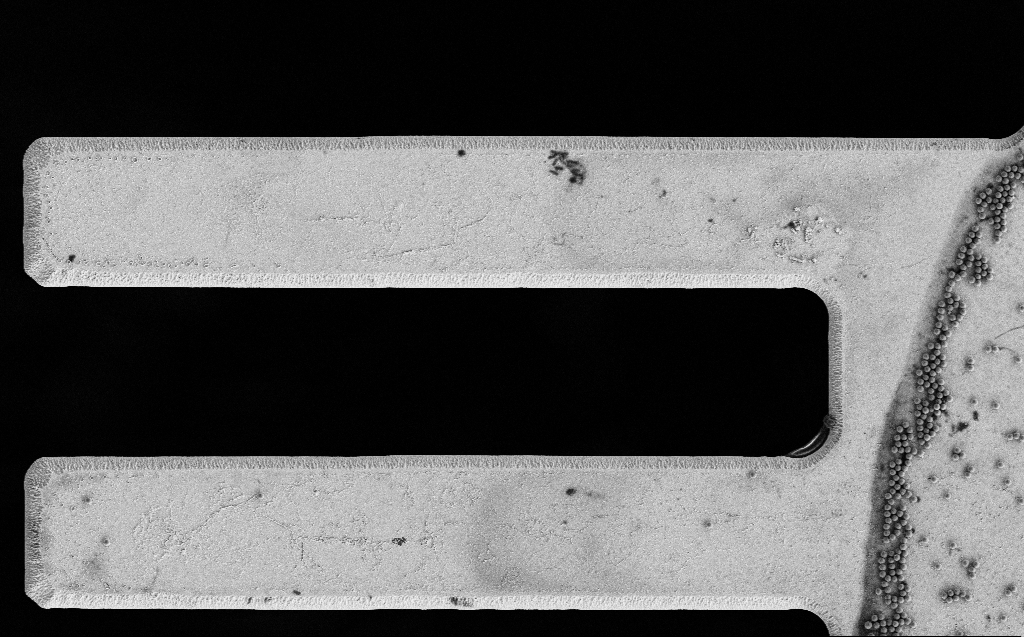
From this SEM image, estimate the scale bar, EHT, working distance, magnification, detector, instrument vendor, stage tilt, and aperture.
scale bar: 20000 nm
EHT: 3 kV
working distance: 7 mm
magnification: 2.96 K X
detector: SE2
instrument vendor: Zeiss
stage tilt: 0°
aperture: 30 µm